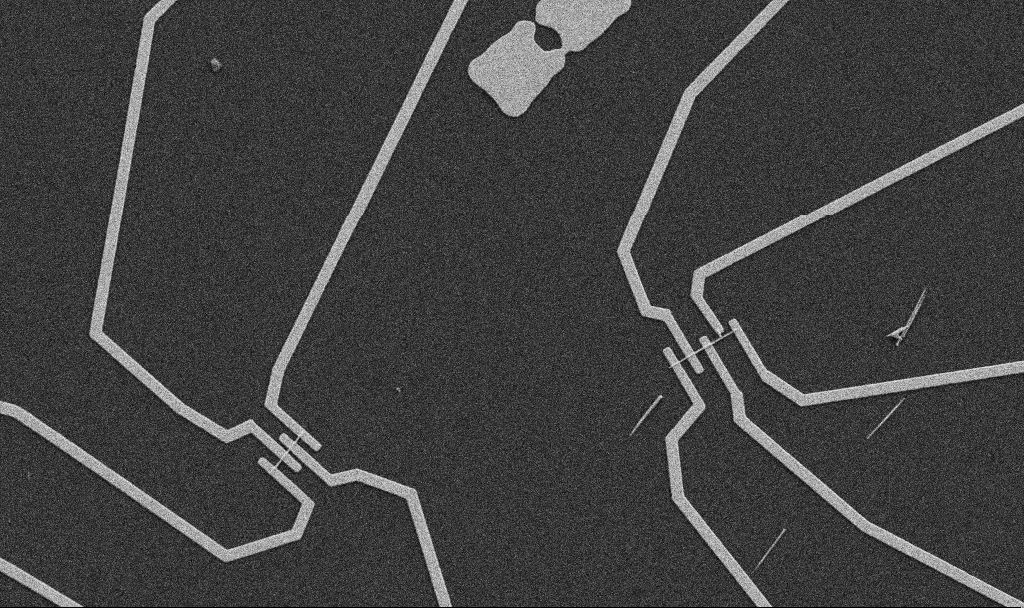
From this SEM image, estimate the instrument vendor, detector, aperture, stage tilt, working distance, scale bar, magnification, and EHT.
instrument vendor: Zeiss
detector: SE2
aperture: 30 µm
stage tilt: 0°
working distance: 10.7 mm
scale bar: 10000 nm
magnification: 5 K X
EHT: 5 kV